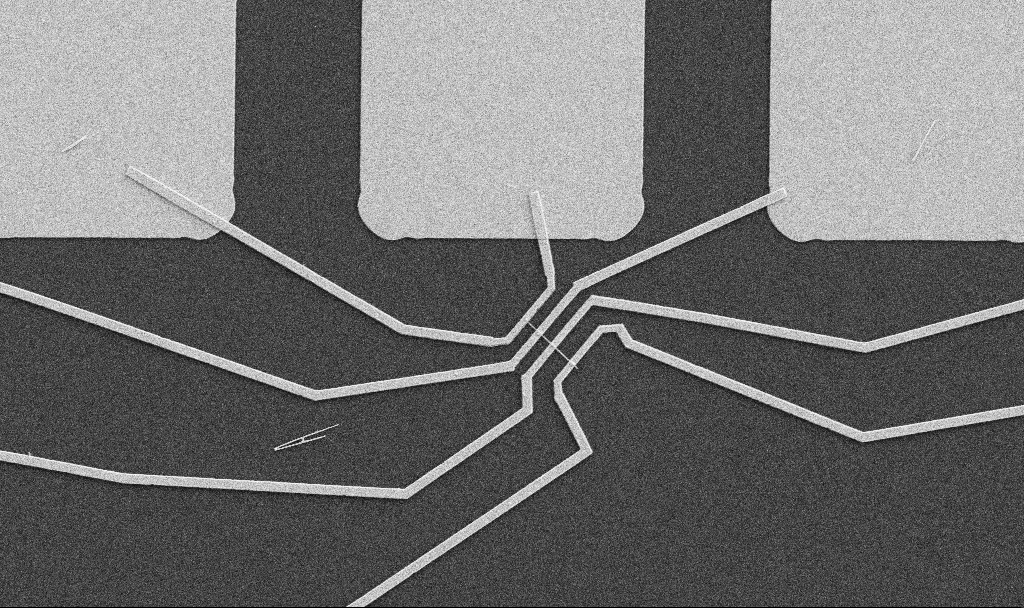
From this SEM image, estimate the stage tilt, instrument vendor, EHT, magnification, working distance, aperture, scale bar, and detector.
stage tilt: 0°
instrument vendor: Zeiss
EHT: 5 kV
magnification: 5 K X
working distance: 10.7 mm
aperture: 30 µm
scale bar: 10000 nm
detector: SE2